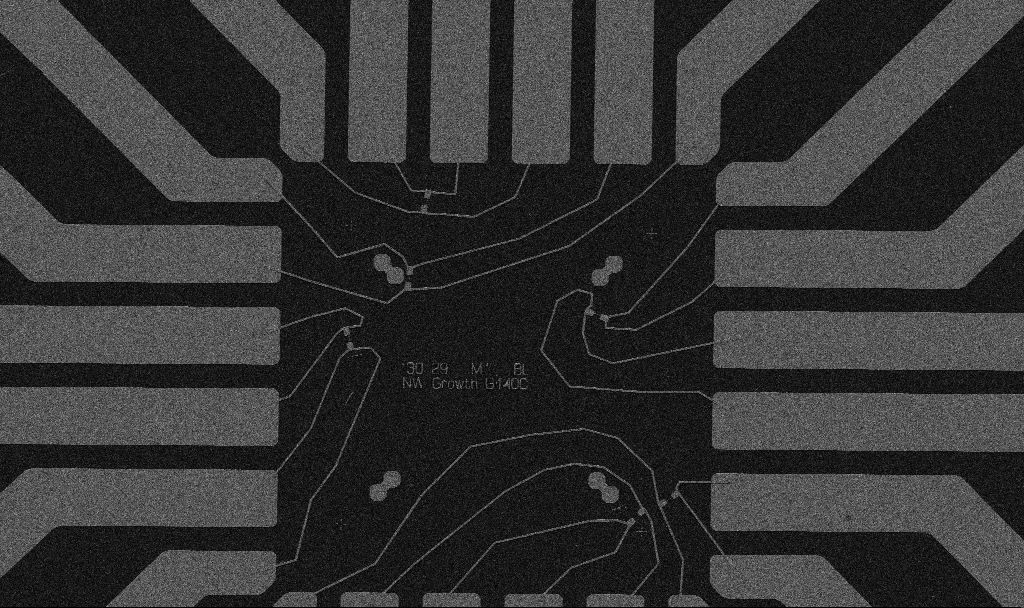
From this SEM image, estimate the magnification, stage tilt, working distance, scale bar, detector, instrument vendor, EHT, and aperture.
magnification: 1 K X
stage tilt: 0°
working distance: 10.7 mm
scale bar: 20000 nm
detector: SE2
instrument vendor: Zeiss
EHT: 5 kV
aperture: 30 µm